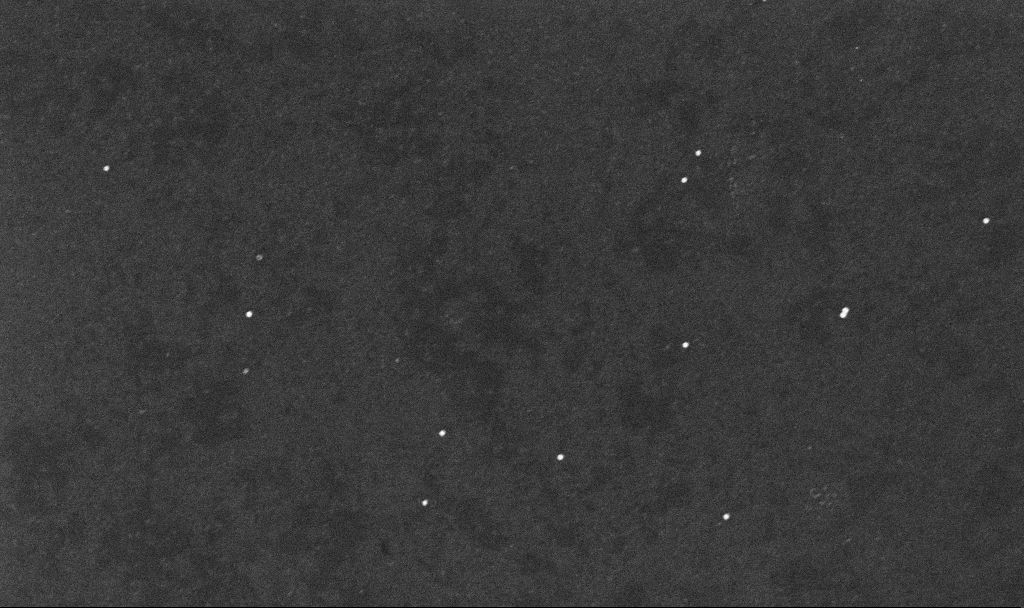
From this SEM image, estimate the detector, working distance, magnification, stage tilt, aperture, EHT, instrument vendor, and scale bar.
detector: InLens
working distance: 3 mm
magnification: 80 K X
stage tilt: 0°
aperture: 30 µm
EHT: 10 kV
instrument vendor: Zeiss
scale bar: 200 nm